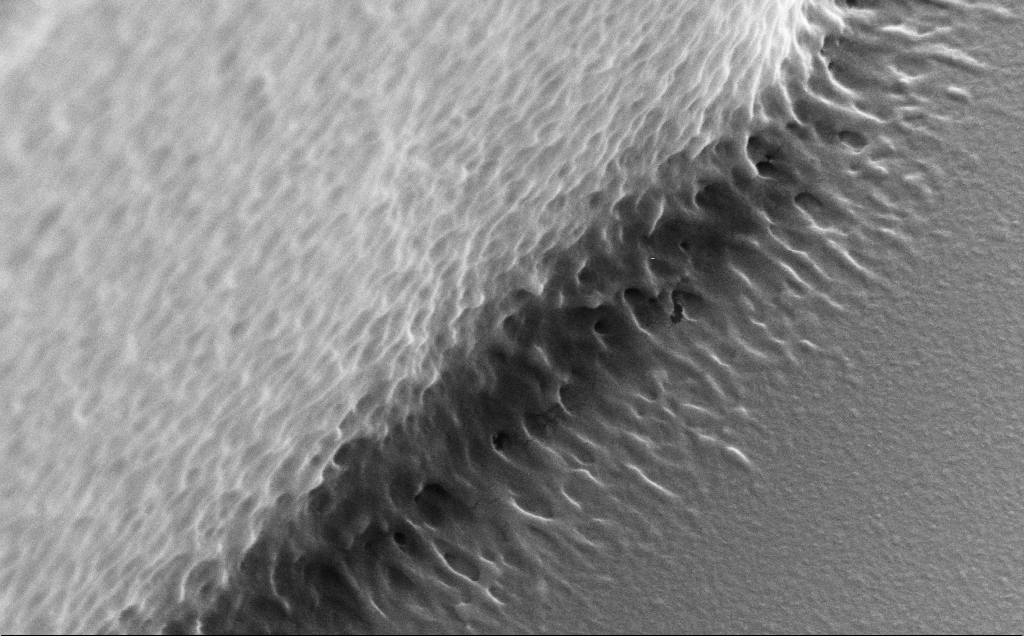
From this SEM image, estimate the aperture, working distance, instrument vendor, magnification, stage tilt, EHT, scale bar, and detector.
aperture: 30 µm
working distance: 11 mm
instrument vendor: Zeiss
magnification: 71.75 K X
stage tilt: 30°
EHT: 5 kV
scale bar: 1000 nm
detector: SE2